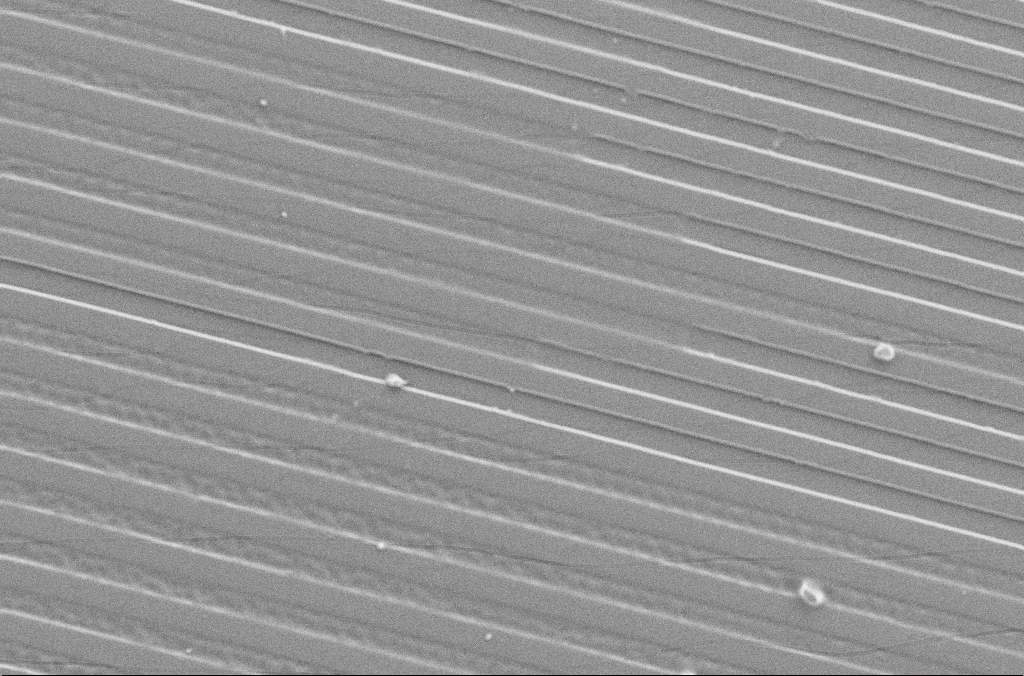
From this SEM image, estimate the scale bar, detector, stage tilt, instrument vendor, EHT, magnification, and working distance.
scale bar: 20000 nm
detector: SE2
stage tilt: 0°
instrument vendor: Zeiss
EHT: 5 kV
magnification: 1 K X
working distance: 4.1 mm